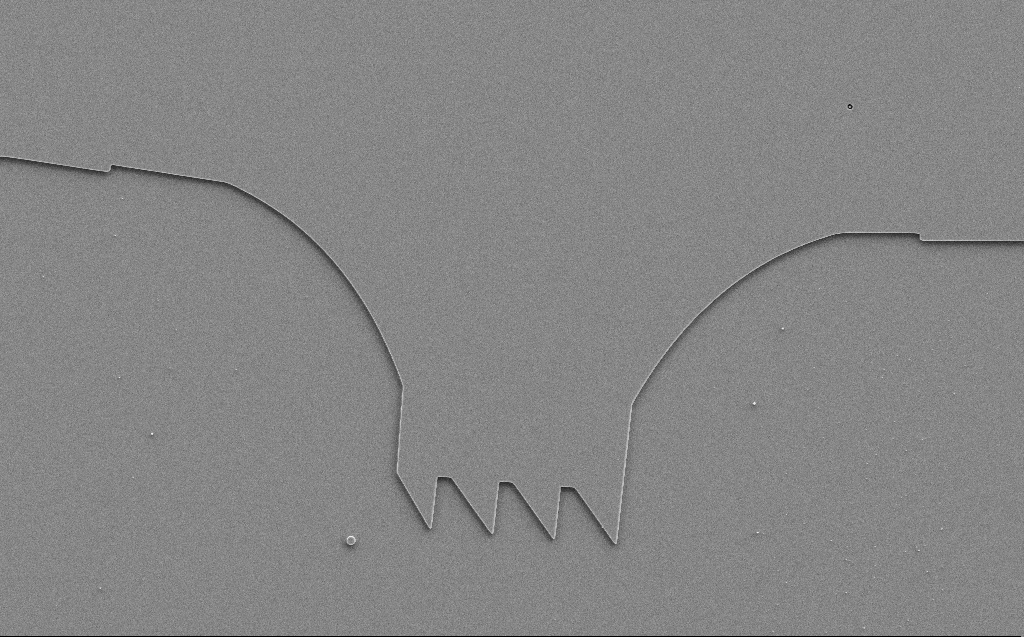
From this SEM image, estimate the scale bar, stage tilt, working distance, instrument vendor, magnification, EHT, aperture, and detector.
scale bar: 20000 nm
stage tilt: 0°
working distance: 6 mm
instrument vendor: Zeiss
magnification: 2.01 K X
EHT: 5 kV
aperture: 30 µm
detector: SE2